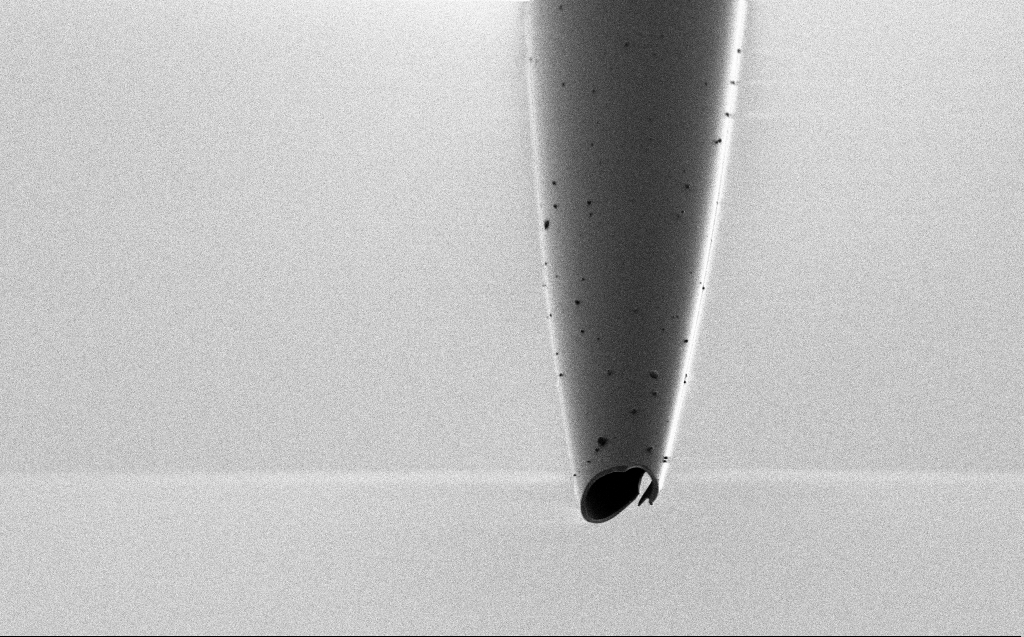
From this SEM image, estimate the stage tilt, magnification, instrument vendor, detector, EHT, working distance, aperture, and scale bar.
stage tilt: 45.1°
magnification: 5.25 K X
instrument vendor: Zeiss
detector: SE2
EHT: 1 kV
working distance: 5 mm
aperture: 30 µm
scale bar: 10000 nm